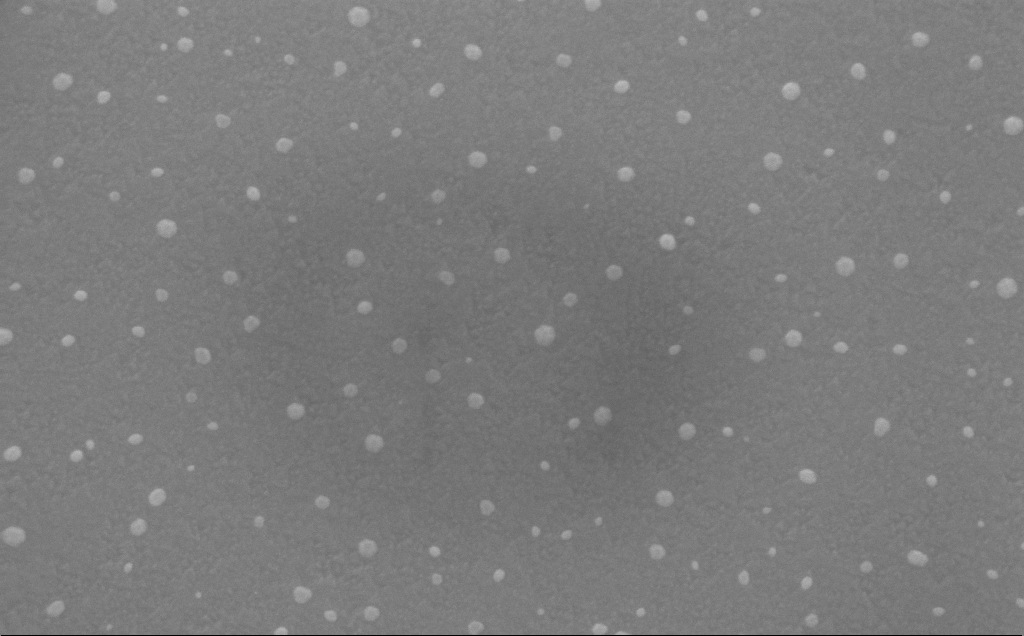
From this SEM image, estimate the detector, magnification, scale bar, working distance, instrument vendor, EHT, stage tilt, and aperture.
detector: InLens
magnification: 127.28 K X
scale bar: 200 nm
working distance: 5 mm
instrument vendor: Zeiss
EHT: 10 kV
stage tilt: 15.5°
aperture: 30 µm